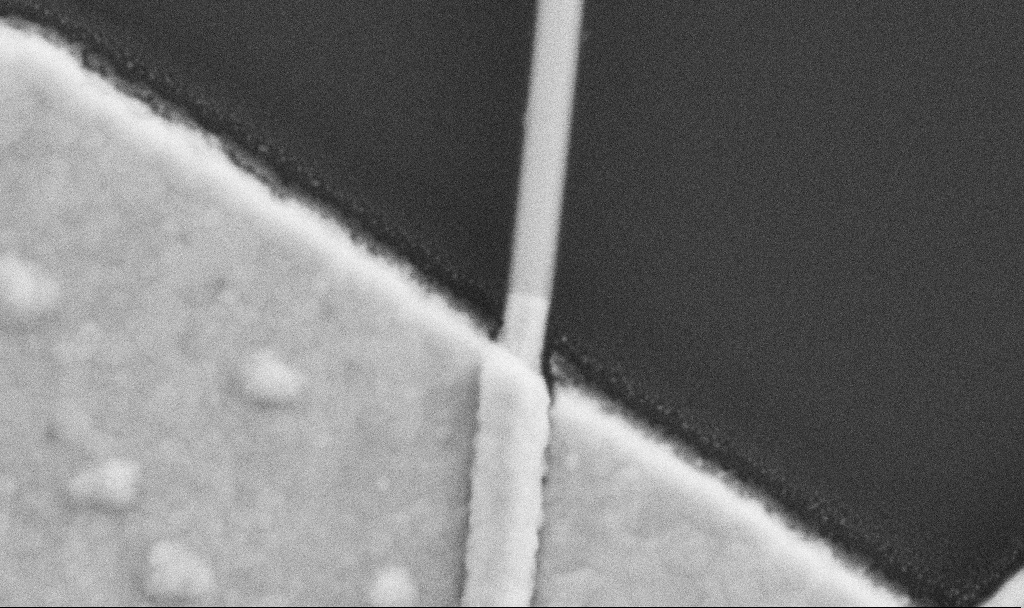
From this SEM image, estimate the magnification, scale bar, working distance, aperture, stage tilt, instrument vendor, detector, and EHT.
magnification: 200 K X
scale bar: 200 nm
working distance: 8.7 mm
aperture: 30 µm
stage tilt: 0°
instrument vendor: Zeiss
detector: SE2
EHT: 5 kV